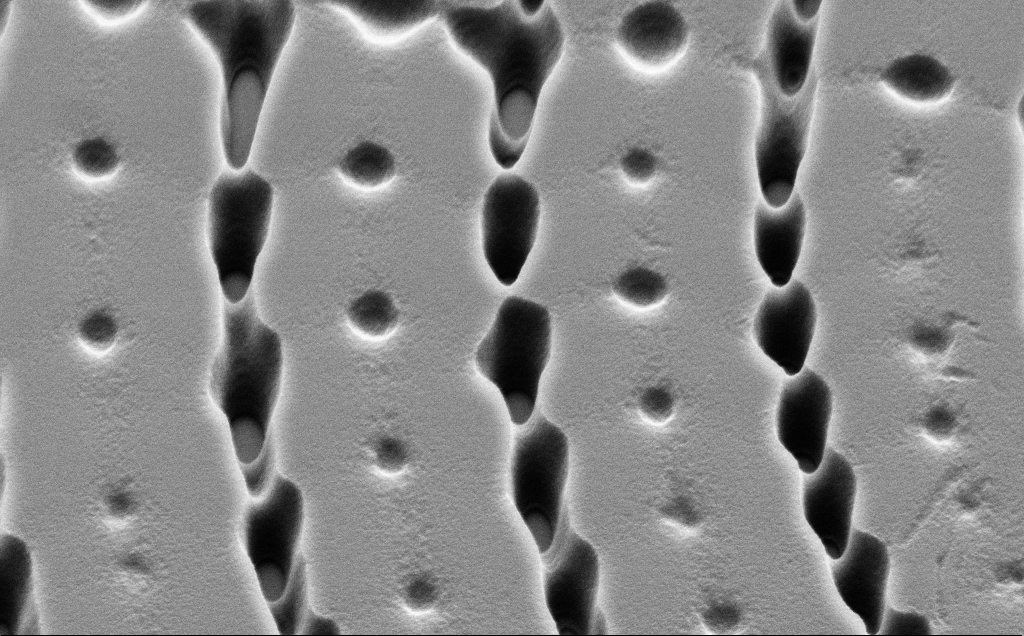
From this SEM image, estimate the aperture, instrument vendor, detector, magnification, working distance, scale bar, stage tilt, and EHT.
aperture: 30 µm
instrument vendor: Zeiss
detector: SE2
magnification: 15.6 K X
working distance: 10 mm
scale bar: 2000 nm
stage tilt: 45°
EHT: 5 kV